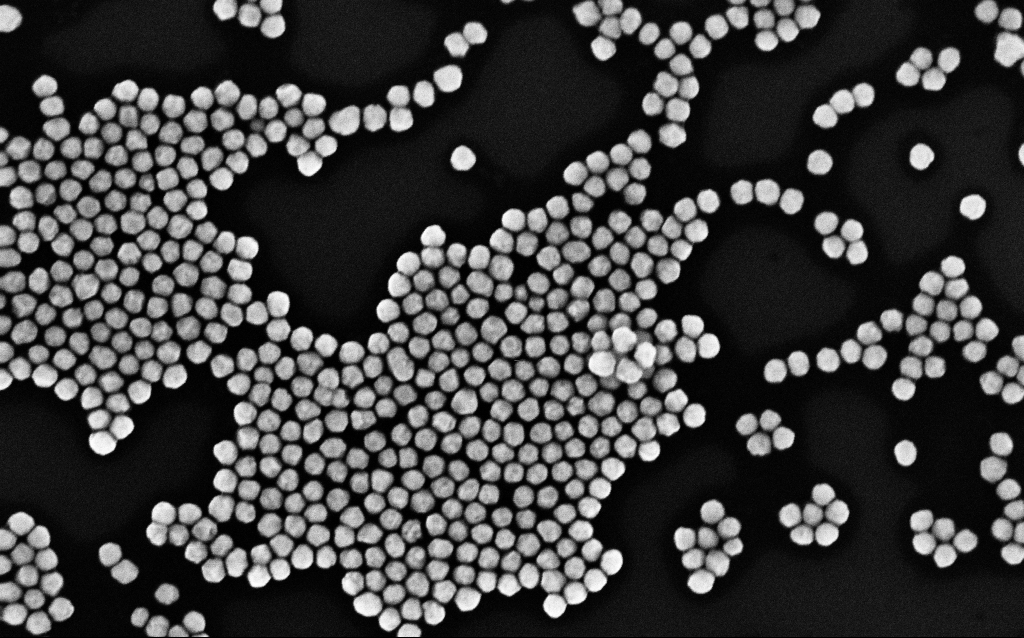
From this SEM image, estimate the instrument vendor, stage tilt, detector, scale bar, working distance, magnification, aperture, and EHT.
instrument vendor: Zeiss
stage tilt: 0°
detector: InLens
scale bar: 200 nm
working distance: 3.1 mm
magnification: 143.25 K X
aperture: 30 µm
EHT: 10 kV